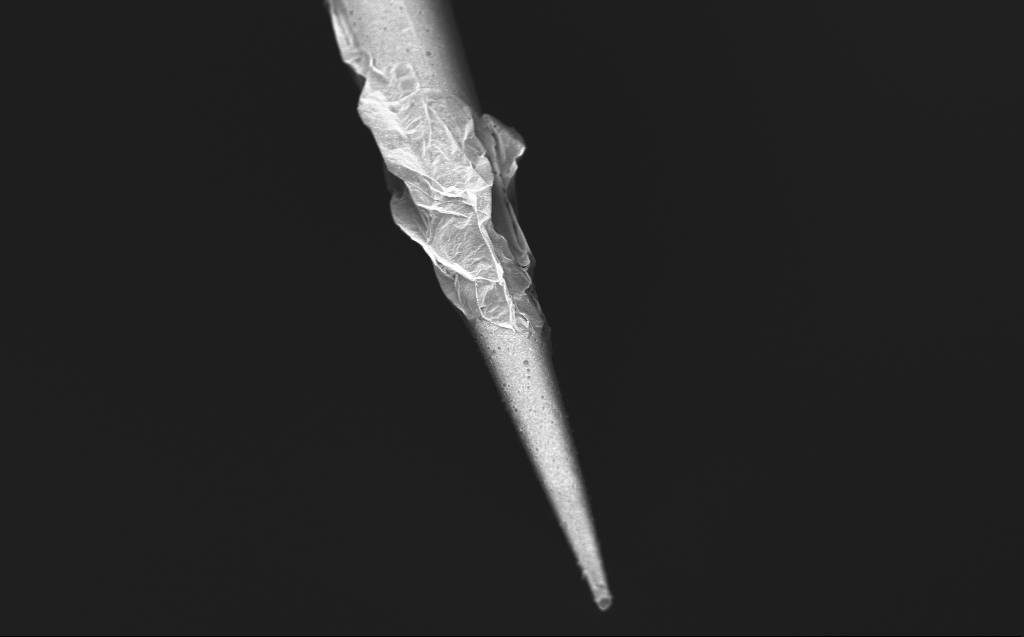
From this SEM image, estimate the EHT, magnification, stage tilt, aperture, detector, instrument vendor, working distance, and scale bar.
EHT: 1 kV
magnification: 10 K X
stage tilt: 45°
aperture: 30 µm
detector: InLens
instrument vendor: Zeiss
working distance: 4 mm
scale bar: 2000 nm